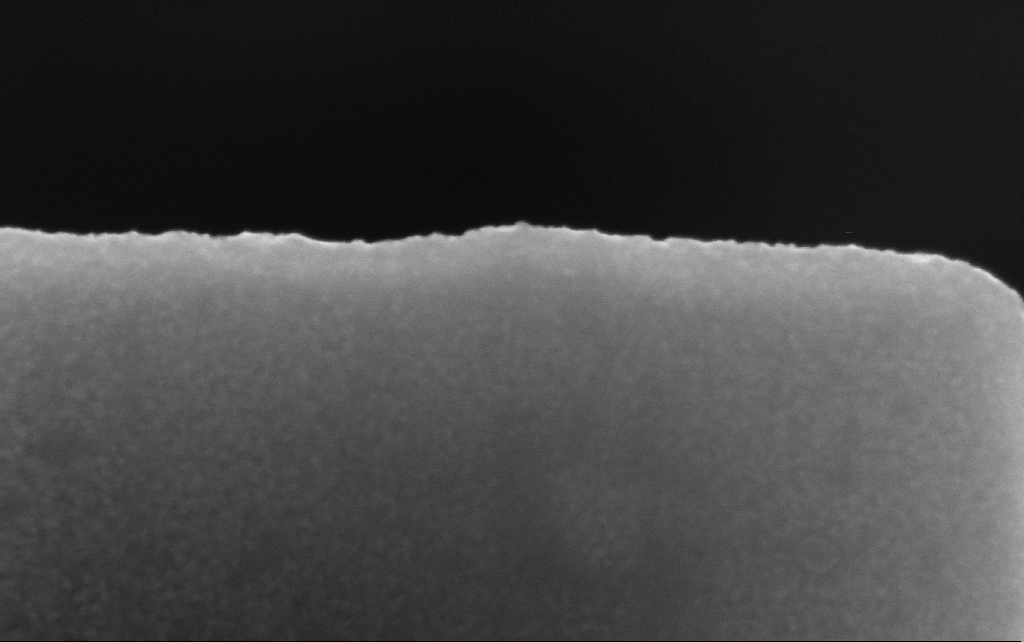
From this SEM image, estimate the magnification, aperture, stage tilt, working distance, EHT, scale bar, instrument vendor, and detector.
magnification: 329.69 K X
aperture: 30 µm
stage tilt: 0°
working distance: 2.5 mm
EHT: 10 kV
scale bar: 100 nm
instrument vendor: Zeiss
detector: InLens